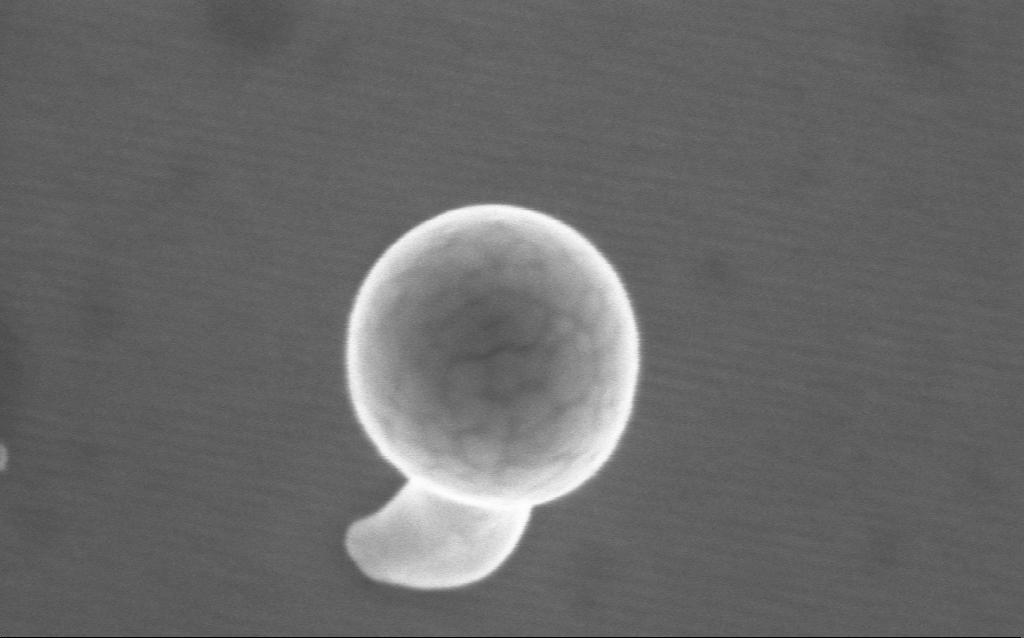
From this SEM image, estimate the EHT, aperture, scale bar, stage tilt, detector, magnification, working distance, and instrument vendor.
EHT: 5 kV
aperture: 30 µm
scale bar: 100 nm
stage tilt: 0°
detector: InLens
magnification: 366.3 K X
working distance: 4 mm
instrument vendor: Zeiss